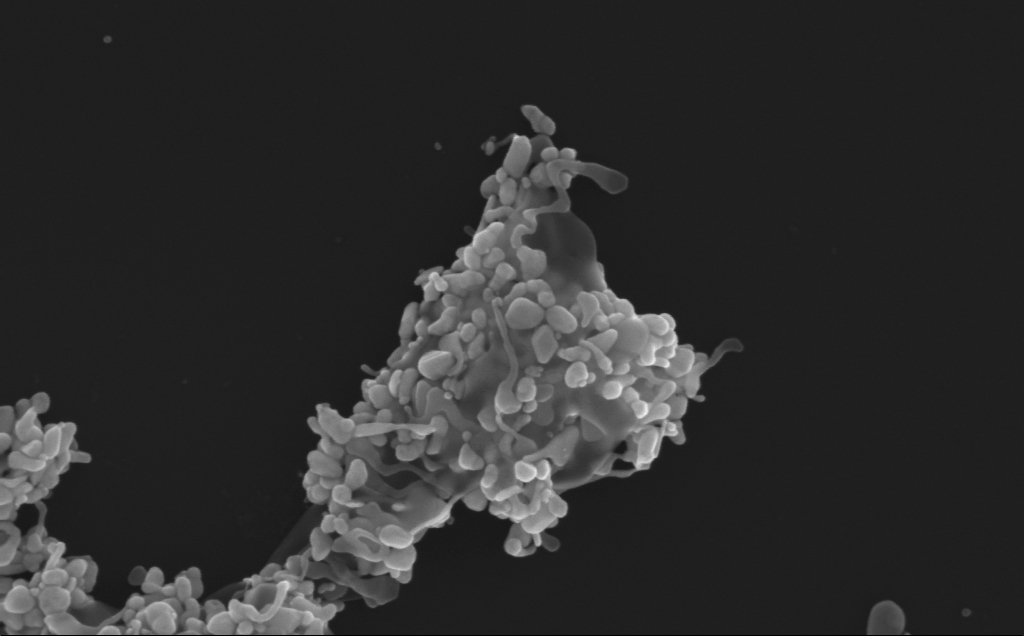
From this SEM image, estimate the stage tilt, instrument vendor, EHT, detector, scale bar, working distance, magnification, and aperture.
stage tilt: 0°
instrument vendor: Zeiss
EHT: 10 kV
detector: InLens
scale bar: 200 nm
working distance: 3 mm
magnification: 147.62 K X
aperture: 30 µm